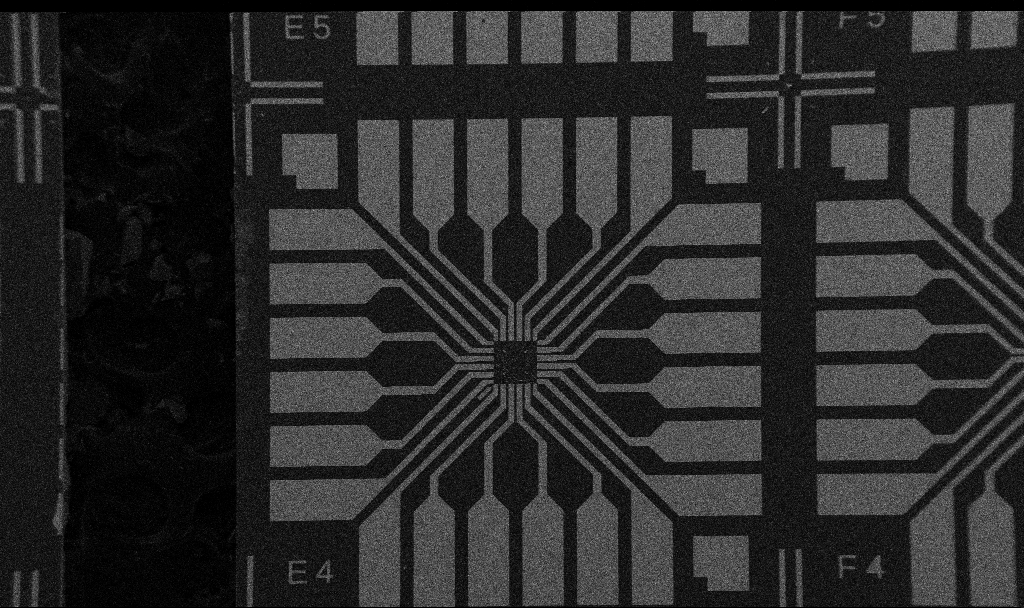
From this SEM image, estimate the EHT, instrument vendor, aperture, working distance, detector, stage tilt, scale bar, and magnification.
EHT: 5 kV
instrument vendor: Zeiss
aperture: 30 µm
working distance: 10.7 mm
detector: SE2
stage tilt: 0°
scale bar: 200000 nm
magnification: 0.1 K X